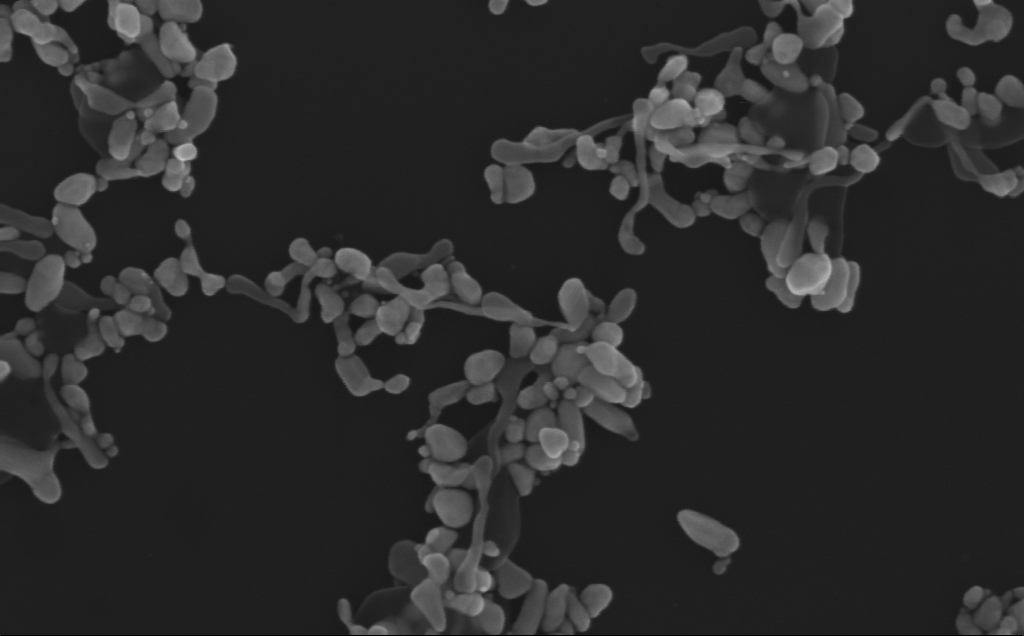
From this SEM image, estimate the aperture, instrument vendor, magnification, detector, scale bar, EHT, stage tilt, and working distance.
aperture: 30 µm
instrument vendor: Zeiss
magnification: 180.36 K X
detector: InLens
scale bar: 200 nm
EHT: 10 kV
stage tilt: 0°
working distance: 3 mm